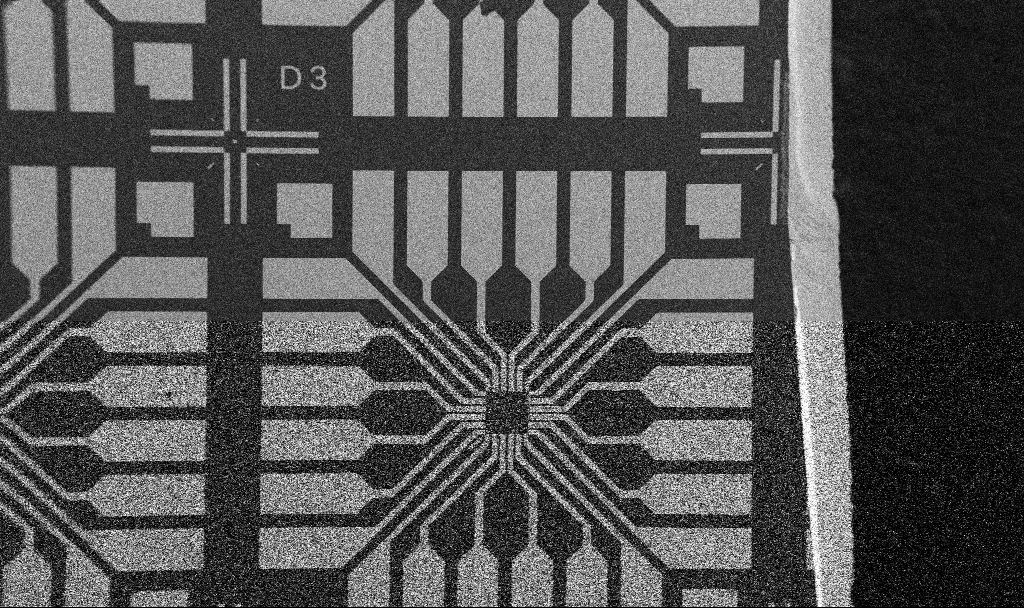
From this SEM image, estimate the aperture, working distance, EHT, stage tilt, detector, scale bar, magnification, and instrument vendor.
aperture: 30 µm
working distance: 10.7 mm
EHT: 5 kV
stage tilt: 0°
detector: SE2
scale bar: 200000 nm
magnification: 0.1 K X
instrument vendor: Zeiss